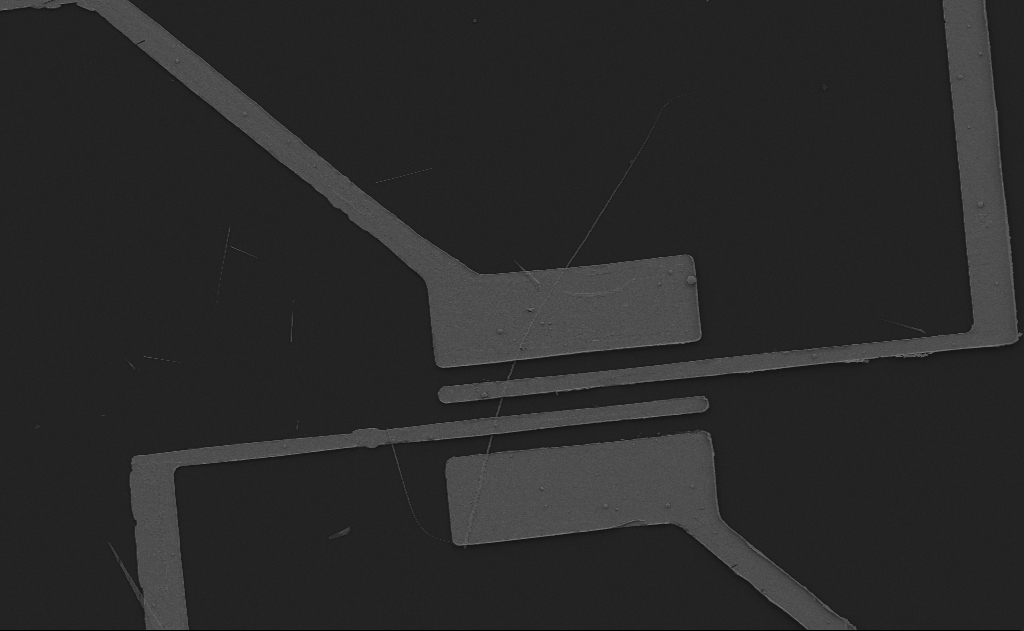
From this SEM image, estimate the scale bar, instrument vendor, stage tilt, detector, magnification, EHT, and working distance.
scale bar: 10000 nm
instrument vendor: Zeiss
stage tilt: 0°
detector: SE2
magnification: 3.29 K X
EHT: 5 kV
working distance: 12 mm